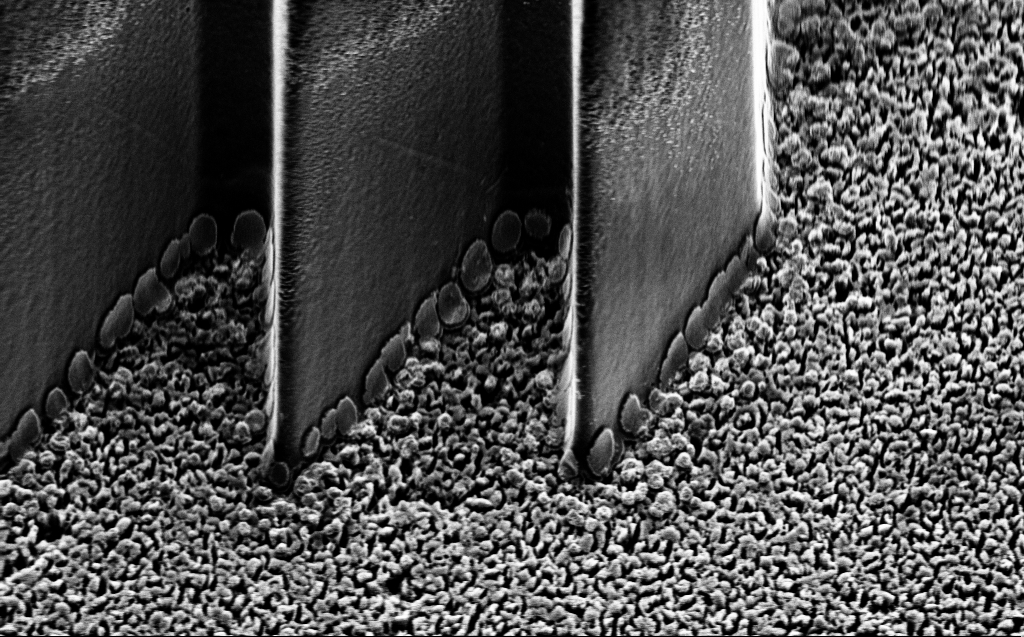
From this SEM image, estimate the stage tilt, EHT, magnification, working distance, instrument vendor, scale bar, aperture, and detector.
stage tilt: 45°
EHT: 5 kV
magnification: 9.57 K X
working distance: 5 mm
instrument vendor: Zeiss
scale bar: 2000 nm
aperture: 30 µm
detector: InLens